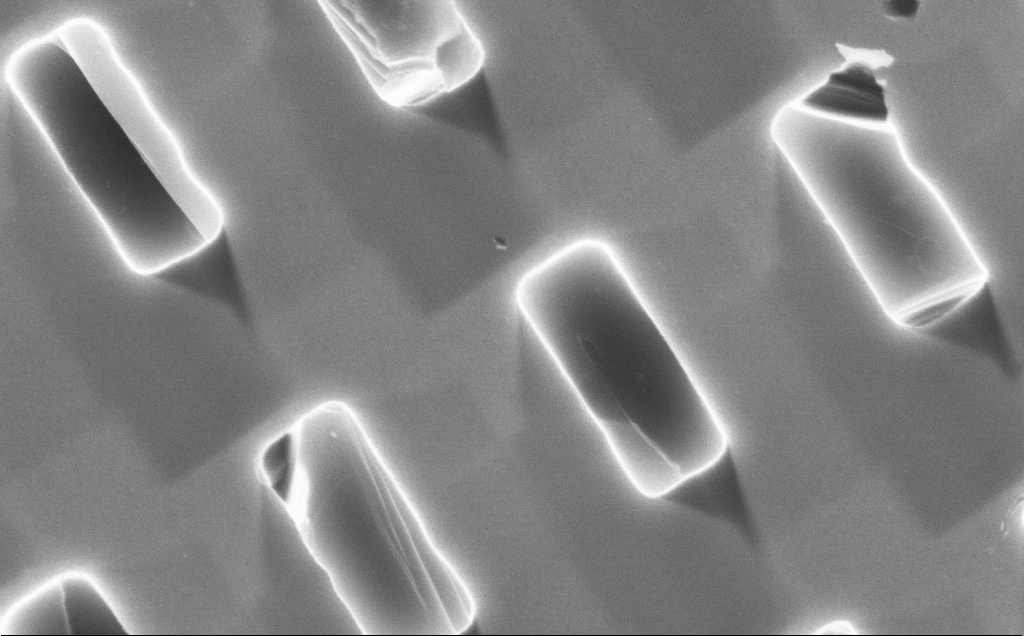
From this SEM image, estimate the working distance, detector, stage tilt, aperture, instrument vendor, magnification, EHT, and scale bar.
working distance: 9 mm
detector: InLens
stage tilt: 0°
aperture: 30 µm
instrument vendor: Zeiss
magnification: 28.32 K X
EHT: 10 kV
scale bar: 2000 nm